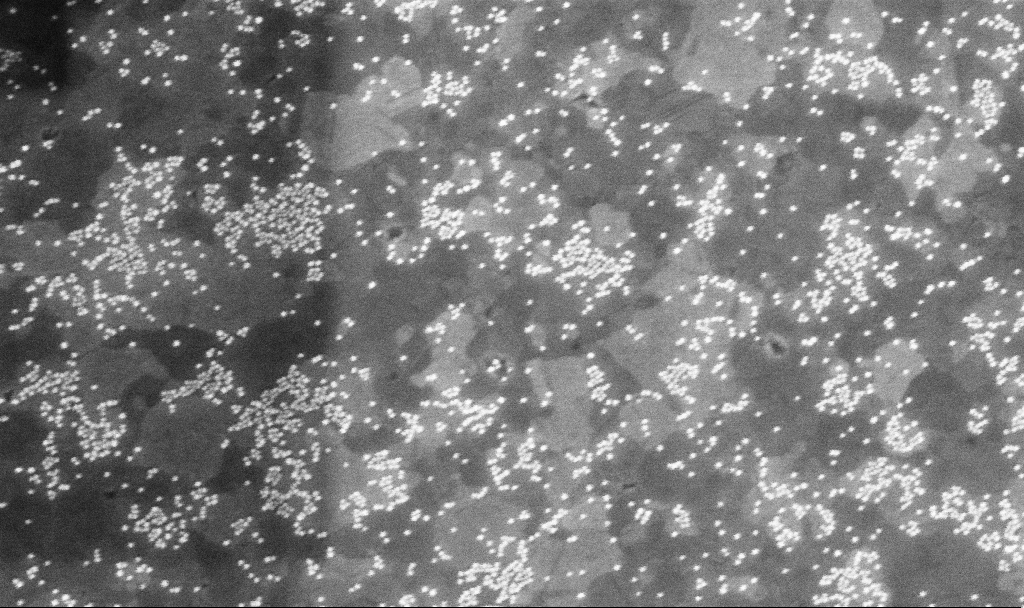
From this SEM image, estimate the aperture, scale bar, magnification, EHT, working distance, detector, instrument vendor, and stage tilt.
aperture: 30 µm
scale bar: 200 nm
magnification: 100 K X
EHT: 5 kV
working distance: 7 mm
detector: InLens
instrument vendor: Zeiss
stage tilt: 0°